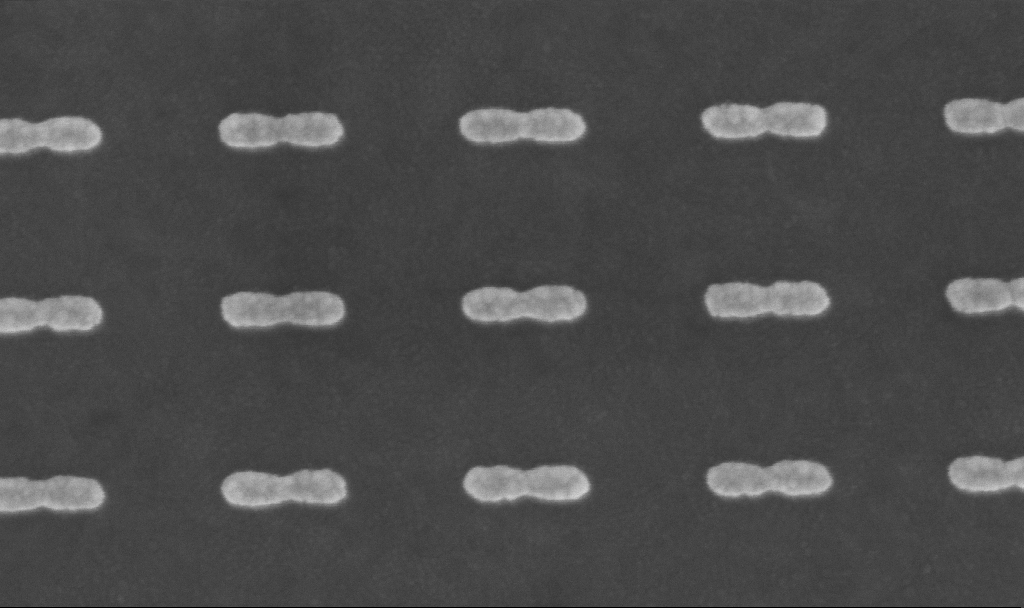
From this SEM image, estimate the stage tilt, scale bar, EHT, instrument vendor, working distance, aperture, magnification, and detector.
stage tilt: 0°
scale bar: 200 nm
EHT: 5 kV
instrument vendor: Zeiss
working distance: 6 mm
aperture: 30 µm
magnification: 219.21 K X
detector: InLens